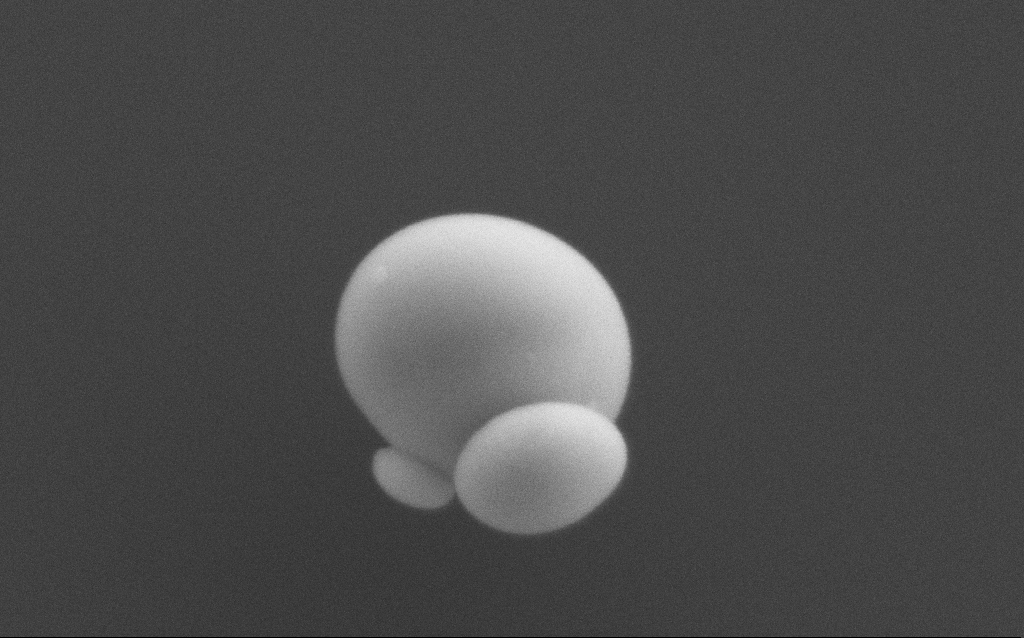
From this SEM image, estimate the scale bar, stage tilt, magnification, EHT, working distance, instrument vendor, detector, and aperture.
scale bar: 200 nm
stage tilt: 0°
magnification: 130.83 K X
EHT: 10 kV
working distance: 4 mm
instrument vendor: Zeiss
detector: SE2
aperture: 30 µm